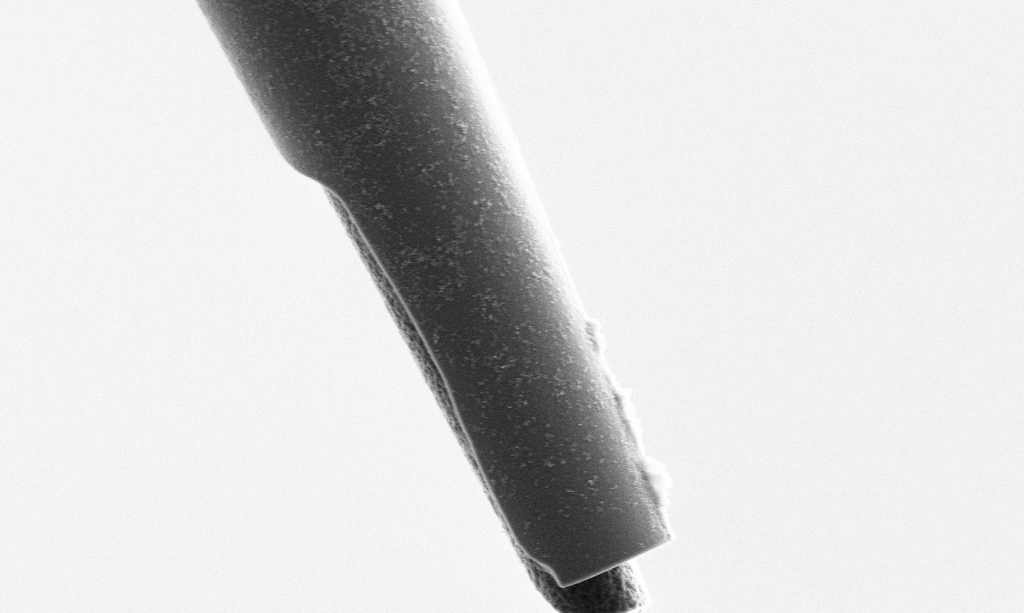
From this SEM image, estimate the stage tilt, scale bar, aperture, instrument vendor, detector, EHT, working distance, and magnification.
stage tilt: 0°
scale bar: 2000 nm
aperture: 30 µm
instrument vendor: Zeiss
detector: SE2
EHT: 3 kV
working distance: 6.5 mm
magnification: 15 K X